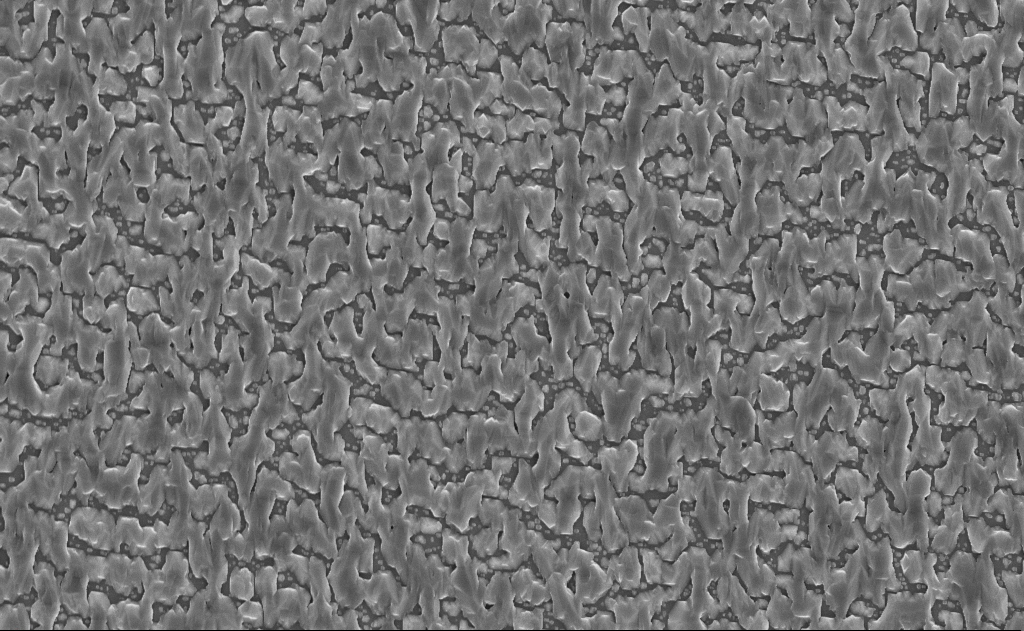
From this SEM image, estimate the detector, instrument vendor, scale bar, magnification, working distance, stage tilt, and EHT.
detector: InLens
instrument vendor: Zeiss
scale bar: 2000 nm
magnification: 10 K X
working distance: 14 mm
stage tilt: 0°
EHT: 10 kV